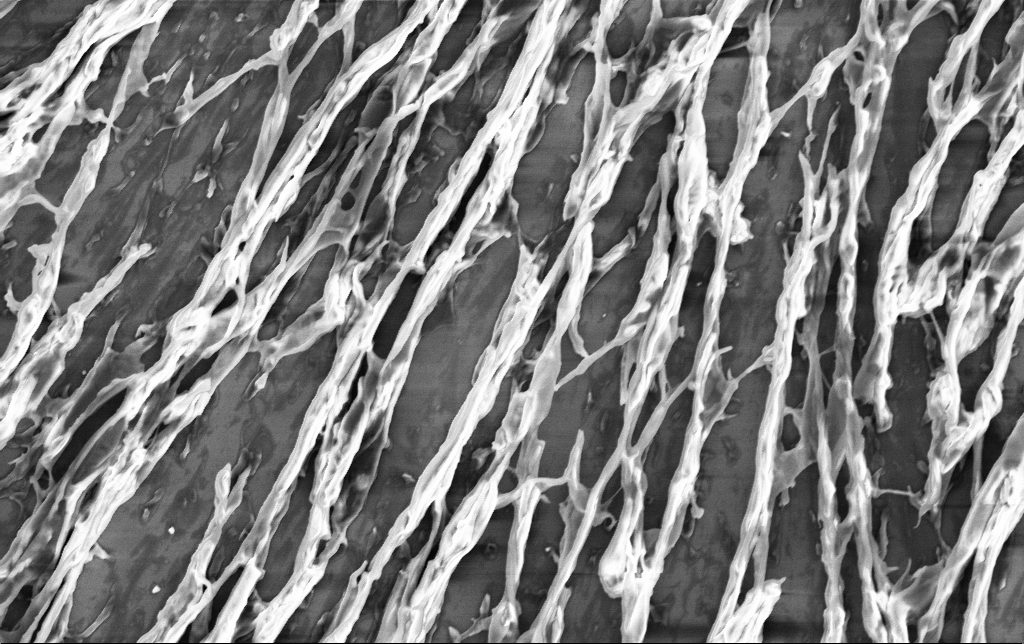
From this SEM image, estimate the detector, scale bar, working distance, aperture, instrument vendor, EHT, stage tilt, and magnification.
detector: InLens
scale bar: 2000 nm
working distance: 3.2 mm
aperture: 30 µm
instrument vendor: Zeiss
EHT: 3 kV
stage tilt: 0°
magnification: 11.88 K X